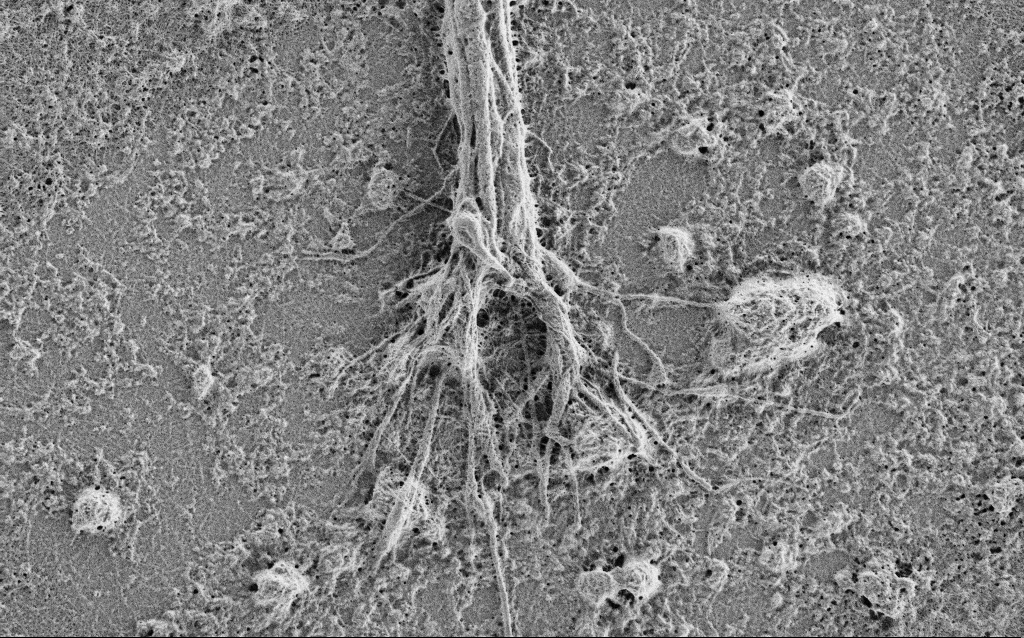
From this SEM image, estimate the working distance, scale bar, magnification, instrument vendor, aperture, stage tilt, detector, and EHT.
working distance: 4 mm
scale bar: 2000 nm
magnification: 10 K X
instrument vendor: Zeiss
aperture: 30 µm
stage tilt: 0°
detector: SE2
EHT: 1 kV